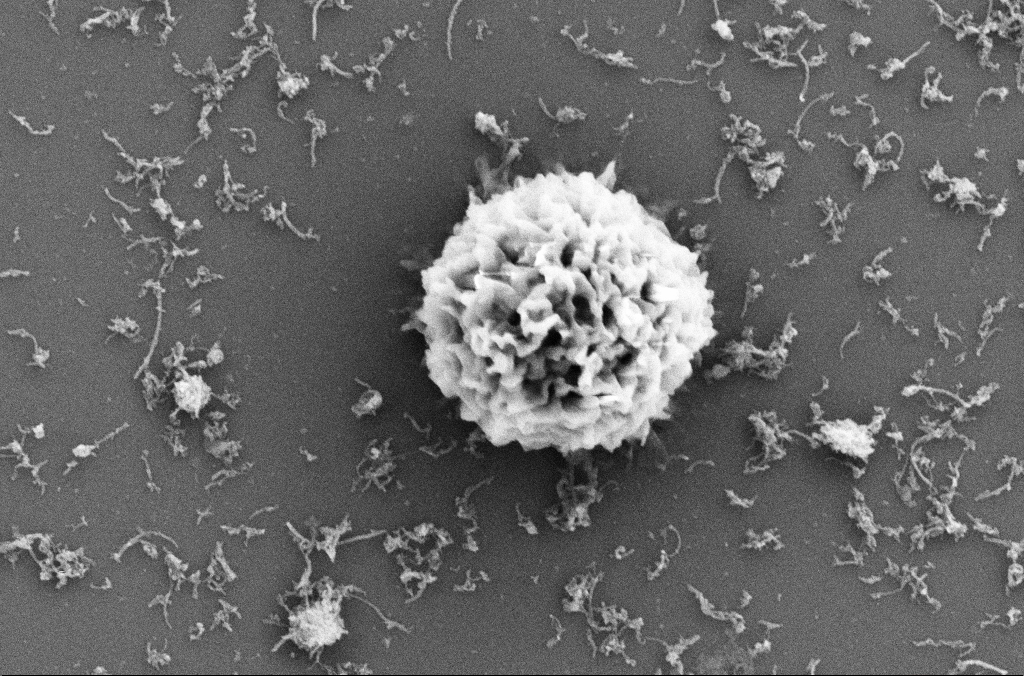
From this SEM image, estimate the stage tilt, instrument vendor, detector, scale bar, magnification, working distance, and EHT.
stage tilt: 0°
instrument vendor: Zeiss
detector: SE2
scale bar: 1000 nm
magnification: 25 K X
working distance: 4 mm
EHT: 5 kV